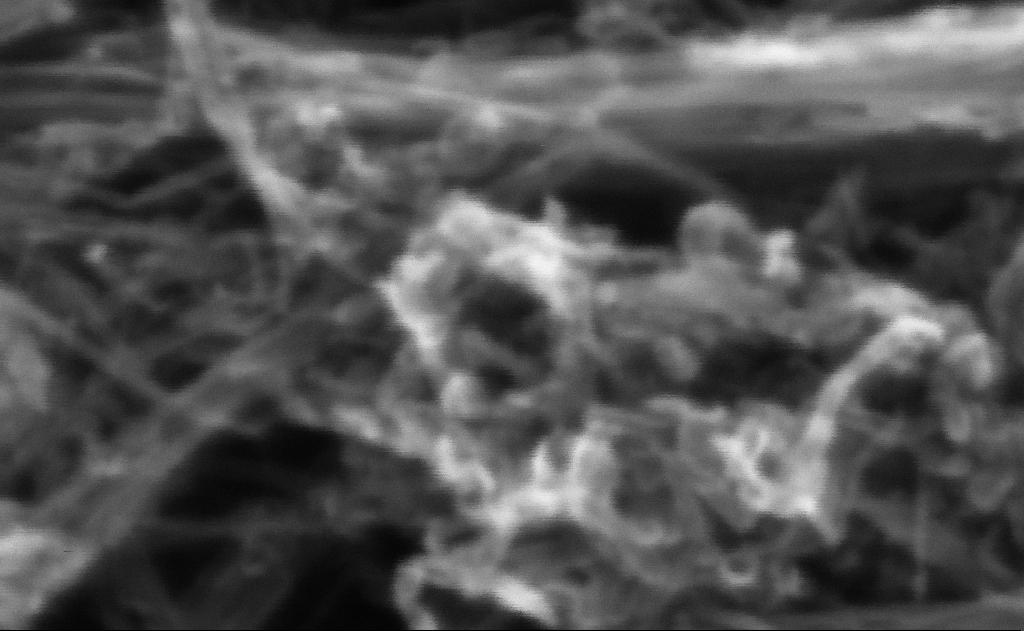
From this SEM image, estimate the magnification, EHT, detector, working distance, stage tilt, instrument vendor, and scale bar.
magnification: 1334.73 K X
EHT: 10 kV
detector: InLens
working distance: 6 mm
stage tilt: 0°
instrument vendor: Zeiss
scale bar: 20 nm